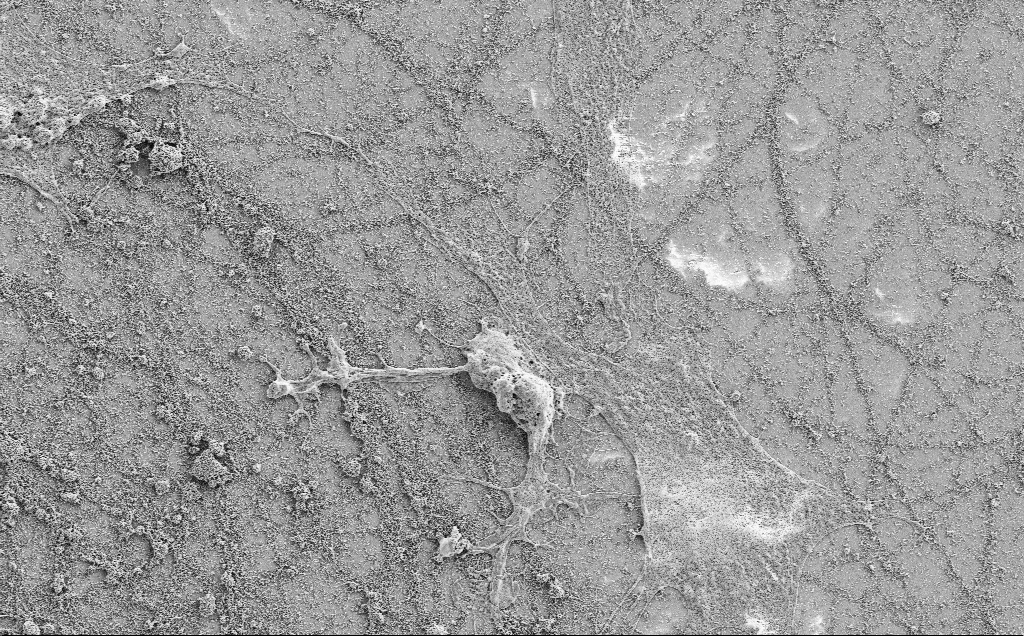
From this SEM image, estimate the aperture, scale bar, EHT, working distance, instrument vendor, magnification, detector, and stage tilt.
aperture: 30 µm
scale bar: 20000 nm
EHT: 2 kV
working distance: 7.1 mm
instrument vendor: Zeiss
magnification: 2.5 K X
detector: SE2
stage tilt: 0°